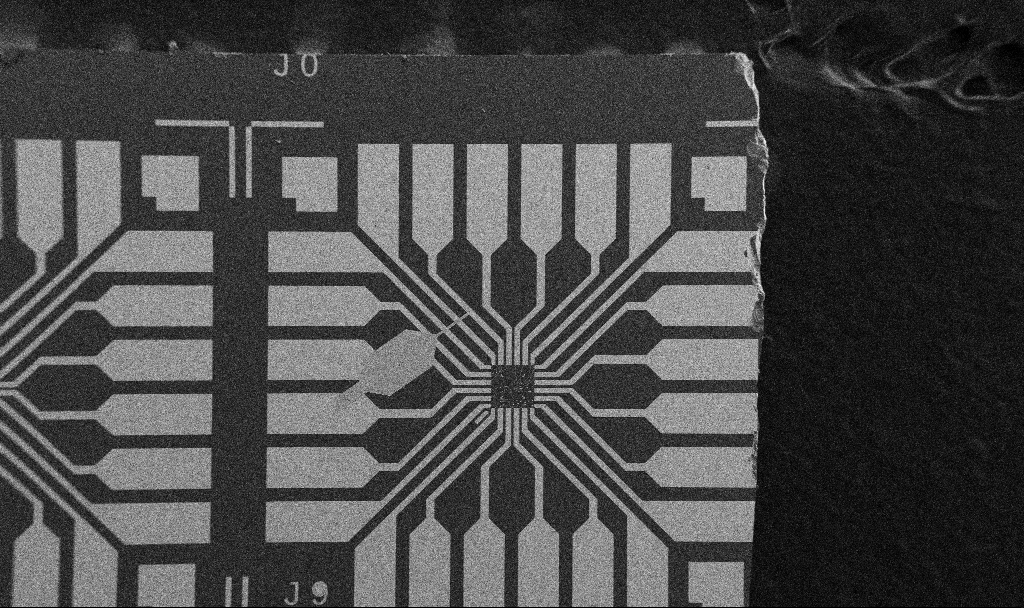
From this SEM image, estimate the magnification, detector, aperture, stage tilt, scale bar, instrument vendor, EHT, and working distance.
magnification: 0.1 K X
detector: SE2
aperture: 30 µm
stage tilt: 0°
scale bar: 200000 nm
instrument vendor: Zeiss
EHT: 5 kV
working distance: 10.7 mm